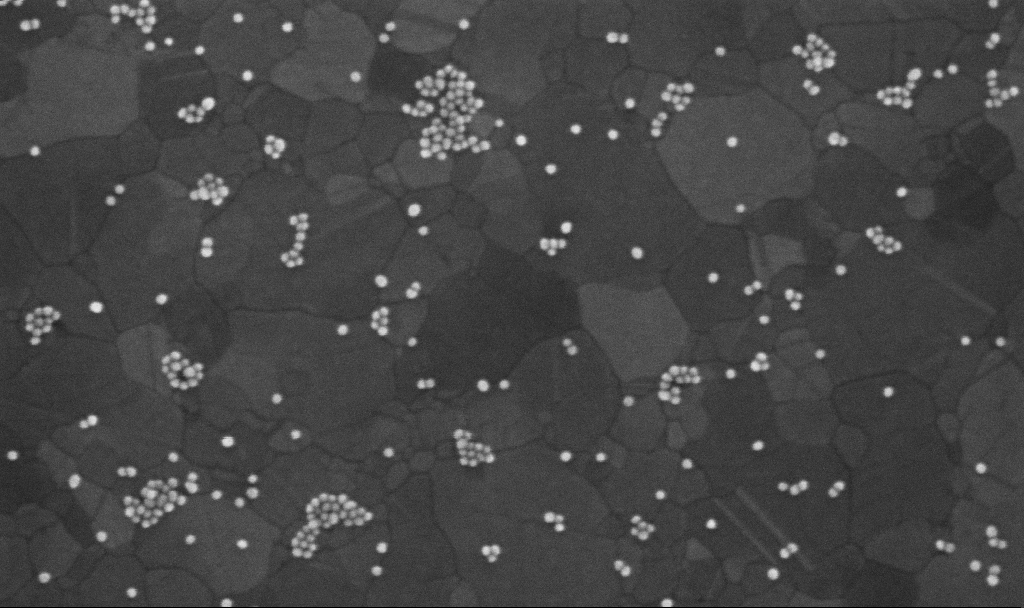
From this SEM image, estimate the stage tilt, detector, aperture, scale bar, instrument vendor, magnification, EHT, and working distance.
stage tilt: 0°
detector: InLens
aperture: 30 µm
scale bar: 200 nm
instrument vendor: Zeiss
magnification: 200 K X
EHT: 10 kV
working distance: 3.8 mm